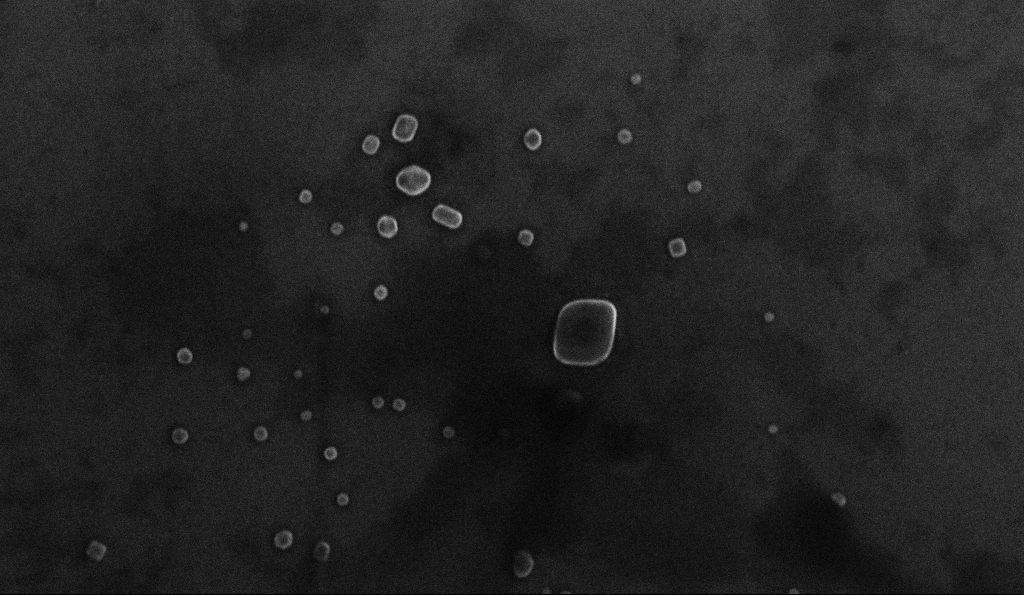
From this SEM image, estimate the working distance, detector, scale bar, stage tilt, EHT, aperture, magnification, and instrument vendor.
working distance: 5.3 mm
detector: InLens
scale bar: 200 nm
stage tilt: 0°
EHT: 10 kV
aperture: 30 µm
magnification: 108.63 K X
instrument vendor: Zeiss